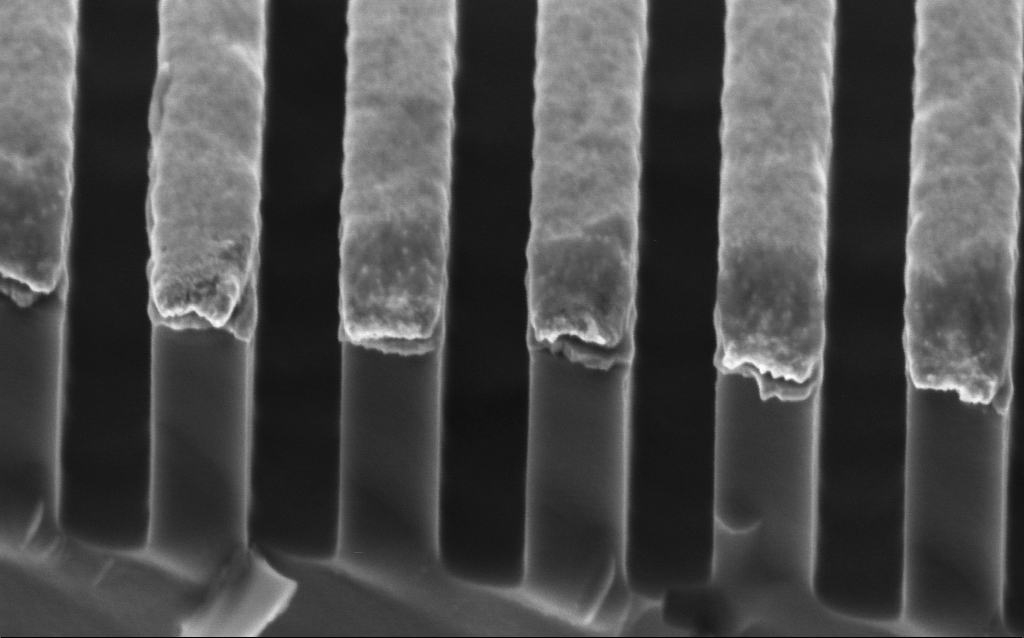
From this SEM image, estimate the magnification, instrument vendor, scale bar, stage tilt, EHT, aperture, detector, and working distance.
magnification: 139.69 K X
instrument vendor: Zeiss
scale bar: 200 nm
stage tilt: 45°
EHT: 2 kV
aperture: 30 µm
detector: InLens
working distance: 2.9 mm